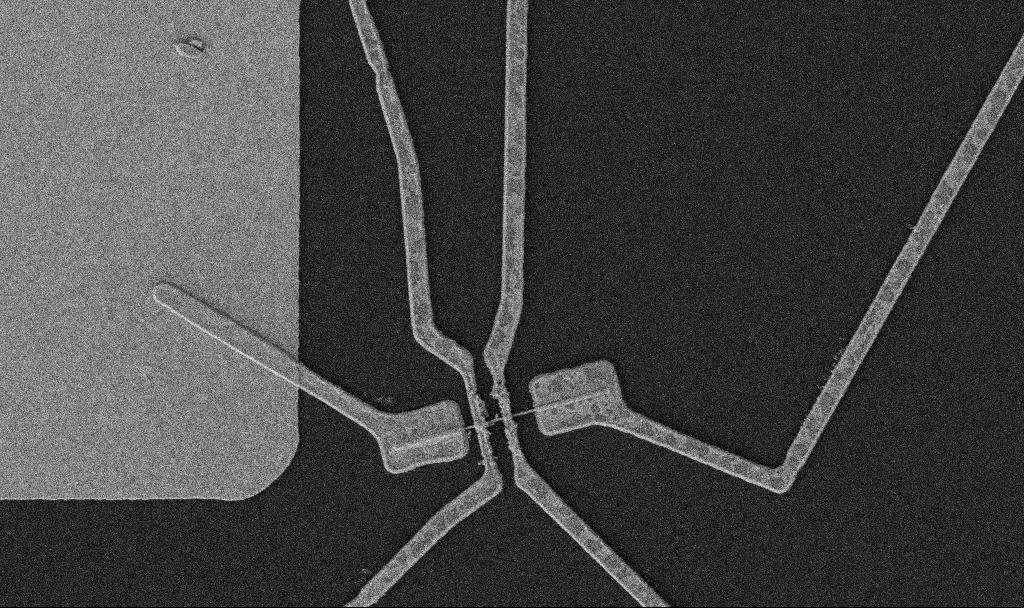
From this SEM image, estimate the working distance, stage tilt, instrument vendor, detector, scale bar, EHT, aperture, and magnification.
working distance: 10.7 mm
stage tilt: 0°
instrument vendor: Zeiss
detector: SE2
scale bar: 2000 nm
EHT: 5 kV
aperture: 30 µm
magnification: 10 K X